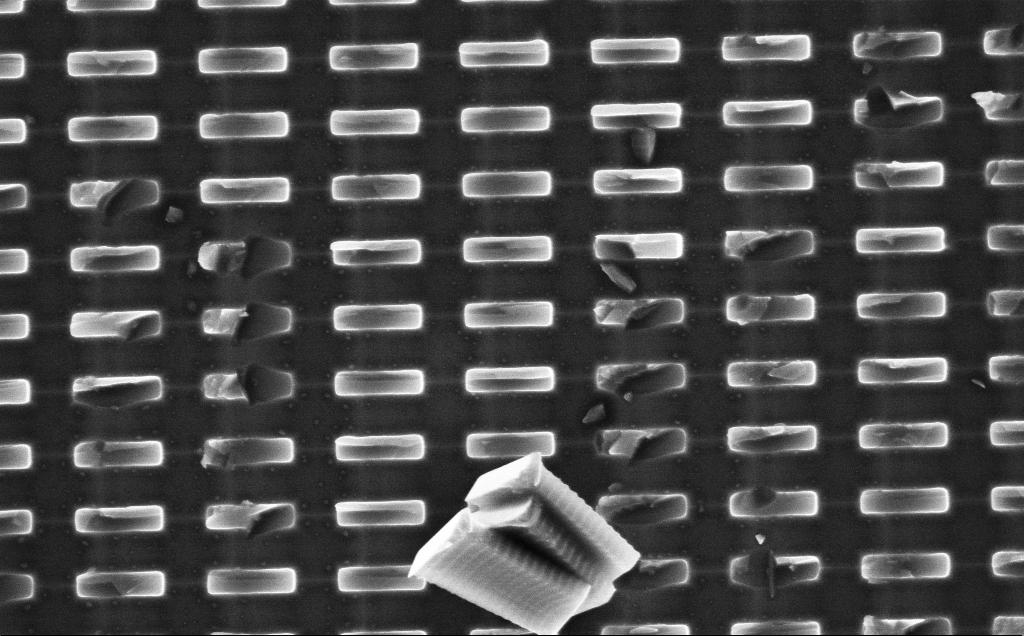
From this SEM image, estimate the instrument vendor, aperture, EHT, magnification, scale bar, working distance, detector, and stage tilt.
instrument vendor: Zeiss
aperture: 30 µm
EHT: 10 kV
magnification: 12.09 K X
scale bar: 1000 nm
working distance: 9 mm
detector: InLens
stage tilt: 0°